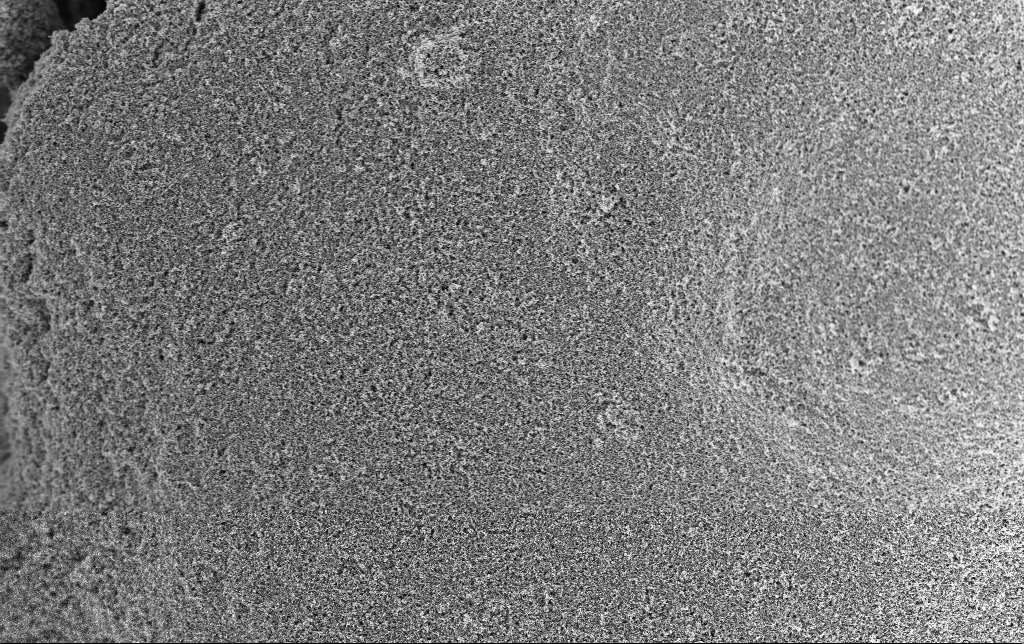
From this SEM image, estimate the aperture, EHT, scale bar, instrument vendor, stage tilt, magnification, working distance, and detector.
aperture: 30 µm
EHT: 3 kV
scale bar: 2000 nm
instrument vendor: Zeiss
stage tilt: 0°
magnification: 10 K X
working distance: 2.8 mm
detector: InLens